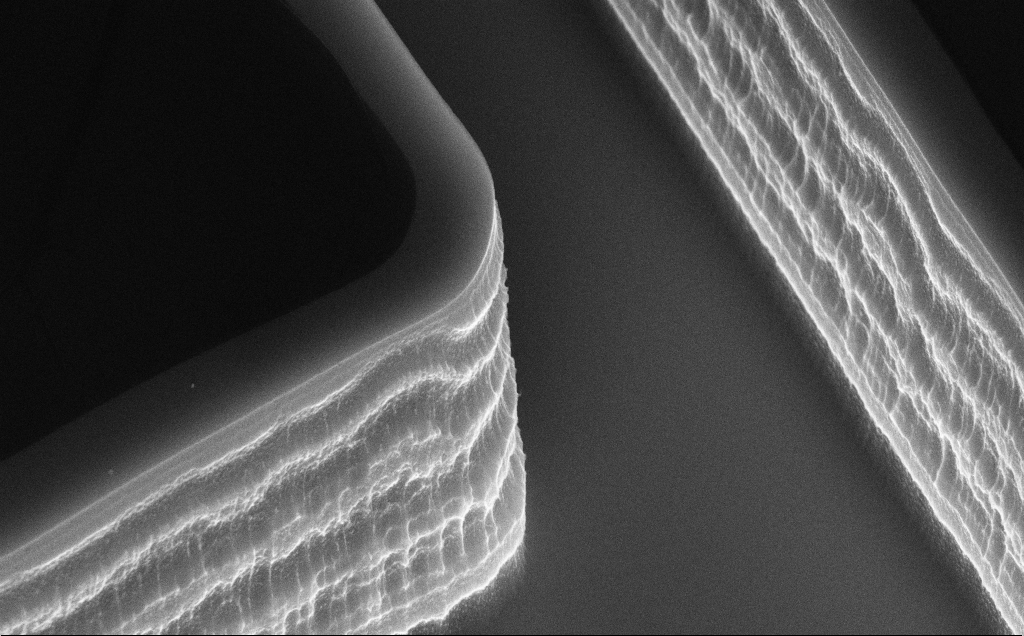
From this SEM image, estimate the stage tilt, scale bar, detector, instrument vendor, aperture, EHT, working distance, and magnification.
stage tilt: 50°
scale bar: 2000 nm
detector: InLens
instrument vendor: Zeiss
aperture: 30 µm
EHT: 10 kV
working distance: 11 mm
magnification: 24.74 K X